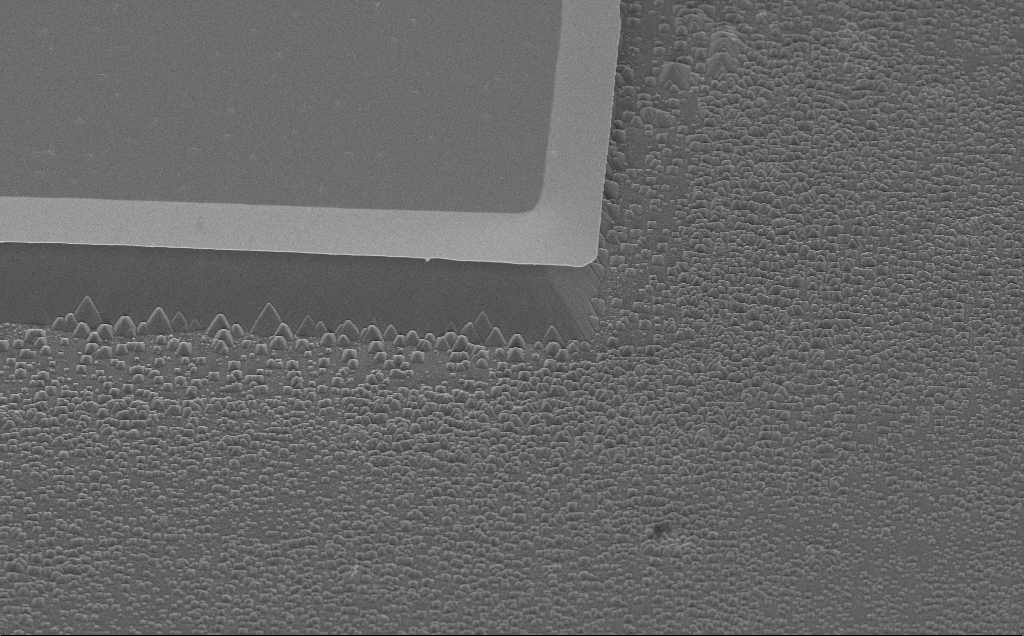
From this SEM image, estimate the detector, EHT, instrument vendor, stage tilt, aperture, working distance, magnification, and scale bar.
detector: InLens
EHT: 5 kV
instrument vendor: Zeiss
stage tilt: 45°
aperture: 30 µm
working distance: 13 mm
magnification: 3.1 K X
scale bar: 20000 nm